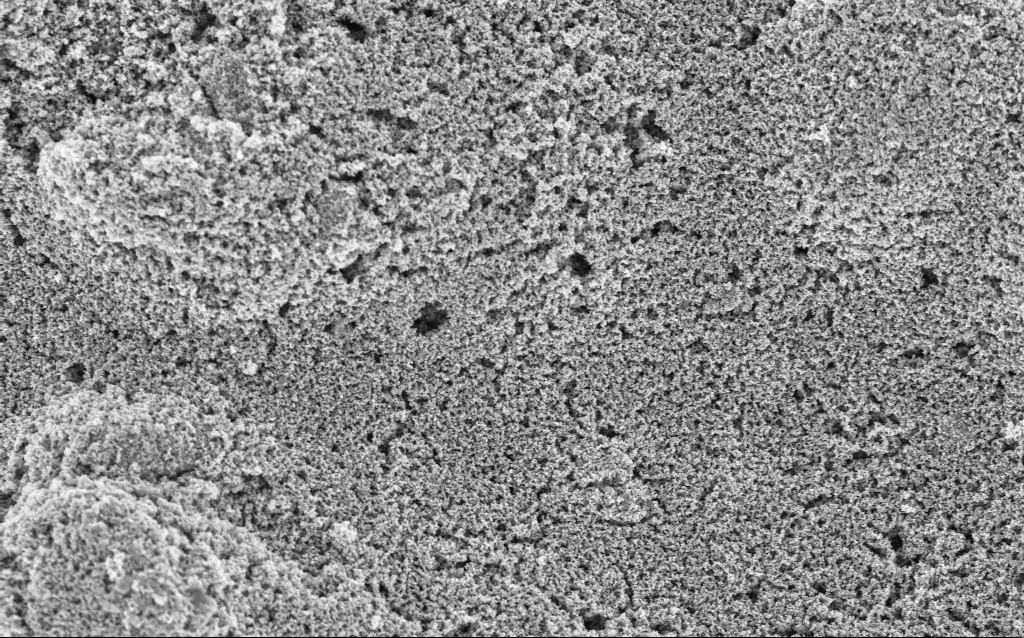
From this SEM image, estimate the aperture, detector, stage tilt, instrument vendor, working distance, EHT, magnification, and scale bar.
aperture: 30 µm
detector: InLens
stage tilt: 0°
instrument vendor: Zeiss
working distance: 7.6 mm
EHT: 3 kV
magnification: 23.9 K X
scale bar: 1000 nm